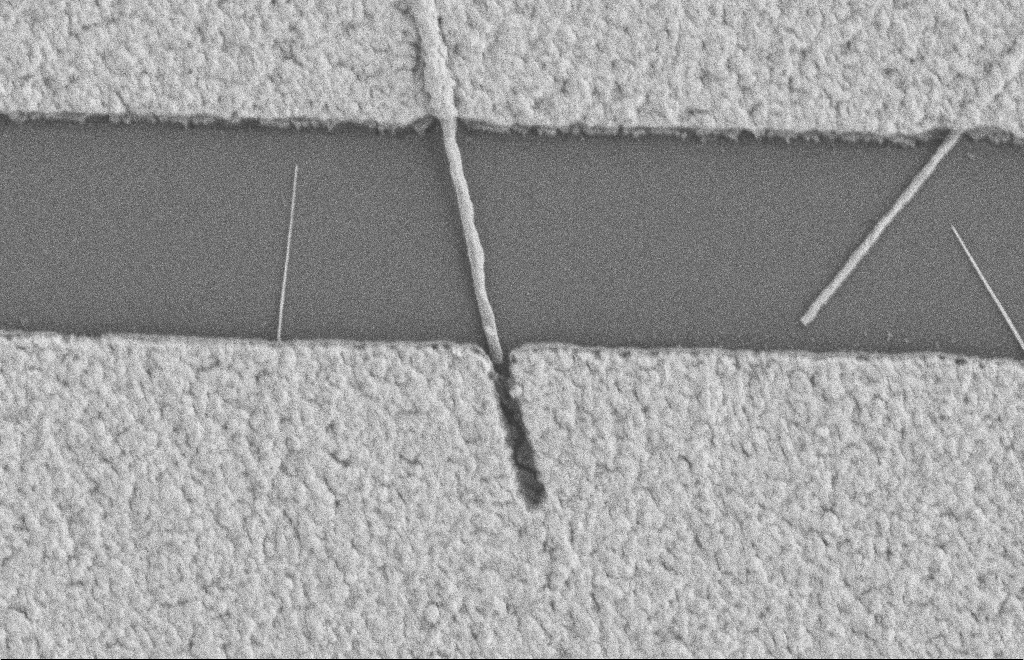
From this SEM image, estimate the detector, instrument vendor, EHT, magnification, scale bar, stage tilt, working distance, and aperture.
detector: SE2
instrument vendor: Zeiss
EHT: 2 kV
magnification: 32.09 K X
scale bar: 1000 nm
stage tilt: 0°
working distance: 8 mm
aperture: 20 µm